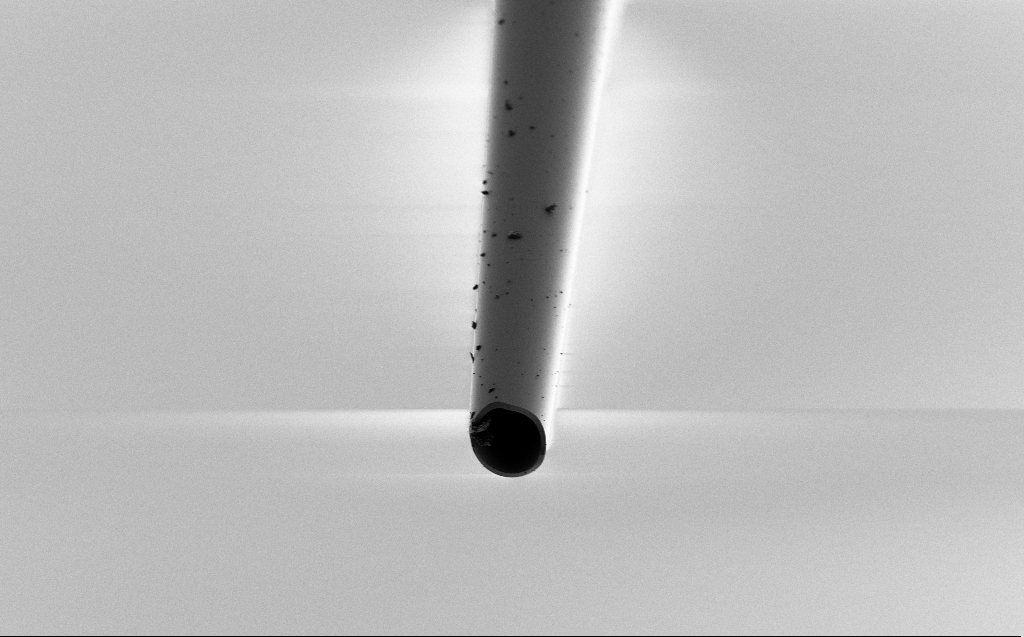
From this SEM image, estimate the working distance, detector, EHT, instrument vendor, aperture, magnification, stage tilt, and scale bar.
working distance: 6 mm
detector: SE2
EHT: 1 kV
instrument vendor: Zeiss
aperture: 30 µm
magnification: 1.61 K X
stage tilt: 45.1°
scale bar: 20000 nm